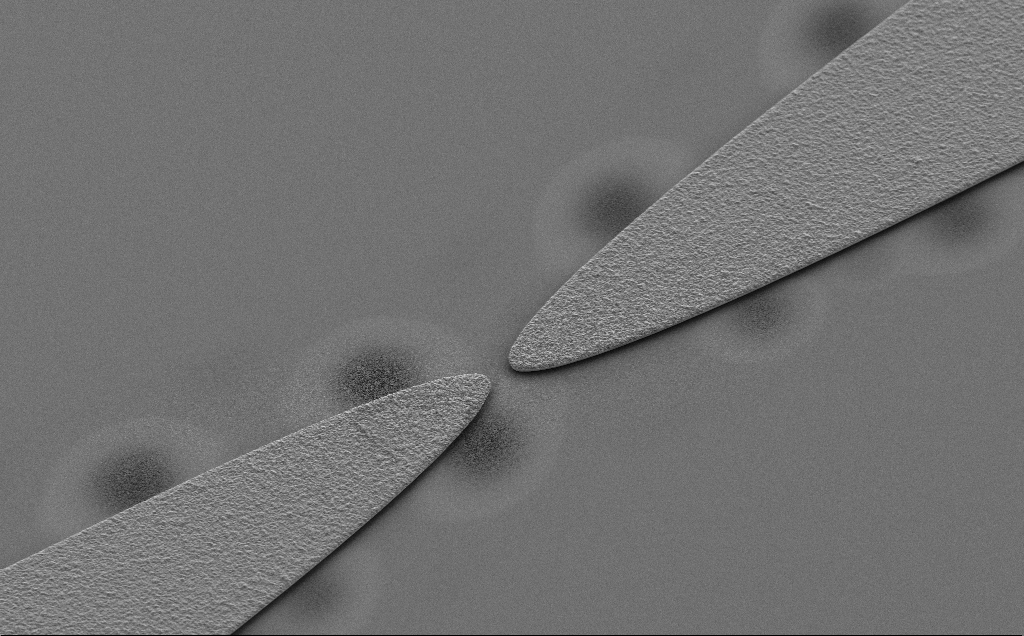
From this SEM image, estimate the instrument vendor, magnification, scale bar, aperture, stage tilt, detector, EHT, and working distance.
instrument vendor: Zeiss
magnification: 1.97 K X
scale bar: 10000 nm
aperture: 30 µm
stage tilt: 30°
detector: SE2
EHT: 5 kV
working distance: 9 mm